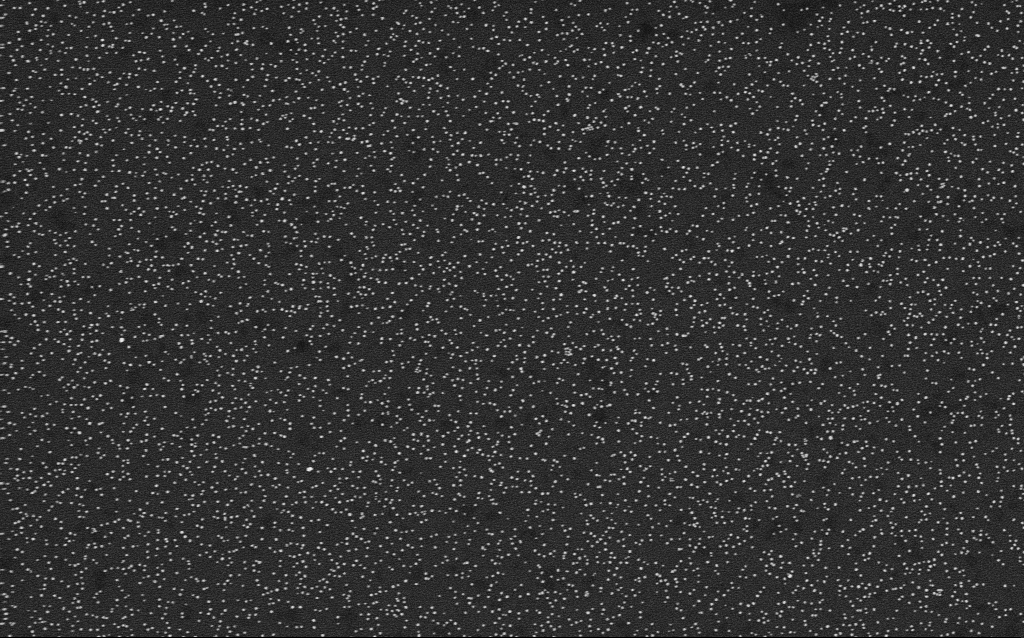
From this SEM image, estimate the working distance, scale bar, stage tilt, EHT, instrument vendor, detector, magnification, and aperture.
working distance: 2.1 mm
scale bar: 1000 nm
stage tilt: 0°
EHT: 4 kV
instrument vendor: Zeiss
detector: InLens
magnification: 50 K X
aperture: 30 µm